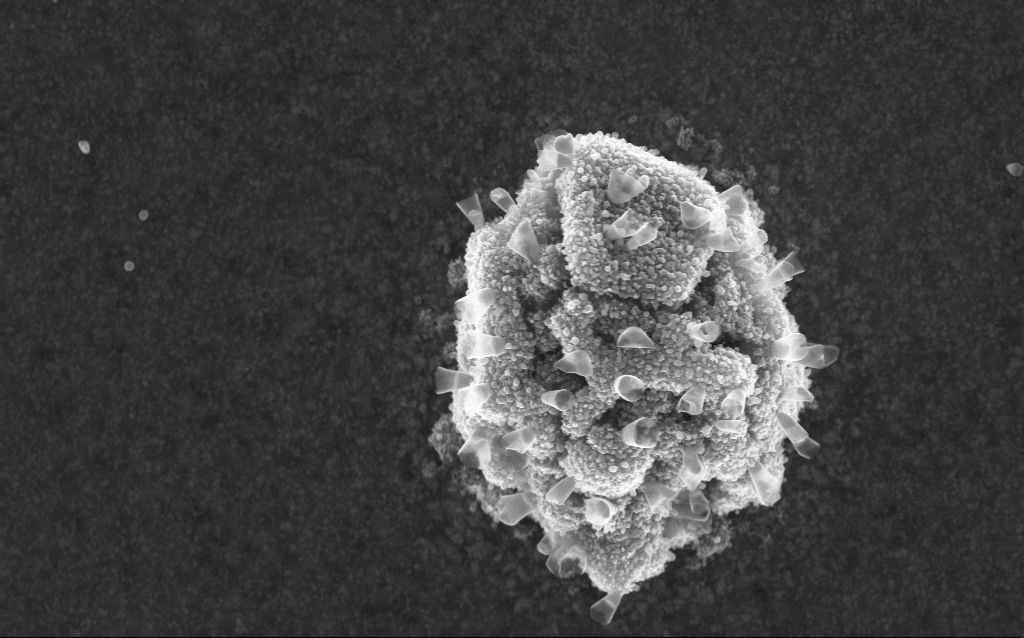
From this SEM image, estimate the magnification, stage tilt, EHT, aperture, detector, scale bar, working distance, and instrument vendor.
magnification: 52.78 K X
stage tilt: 0°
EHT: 5 kV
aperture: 30 µm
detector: InLens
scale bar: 1000 nm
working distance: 2.6 mm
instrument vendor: Zeiss